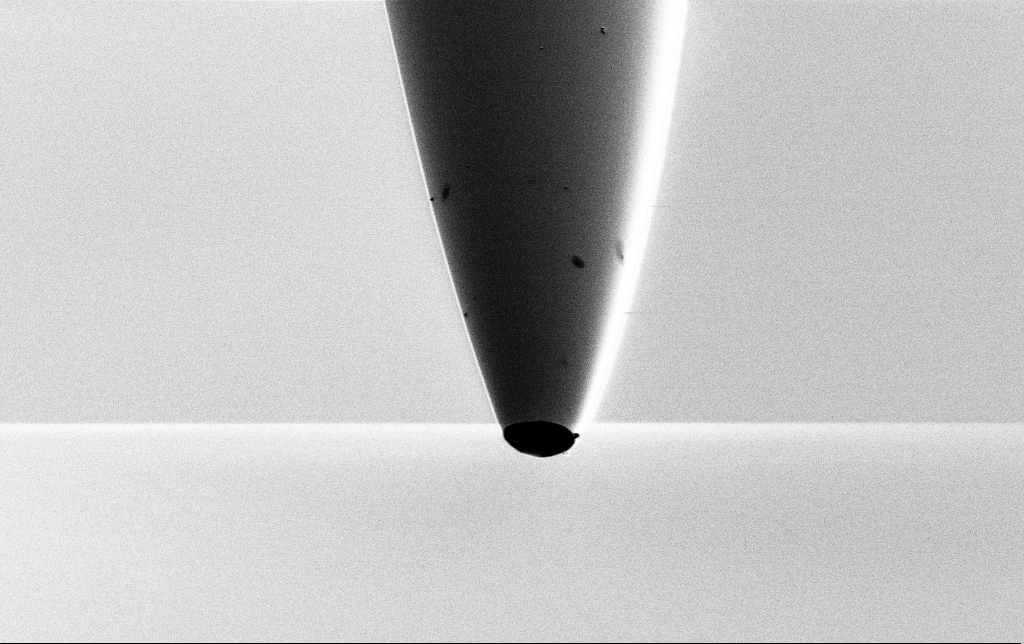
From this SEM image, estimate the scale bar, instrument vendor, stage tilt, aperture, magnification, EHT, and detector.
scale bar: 2000 nm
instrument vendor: Zeiss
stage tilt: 45°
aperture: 30 µm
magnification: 10 K X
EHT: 1 kV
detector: SE2